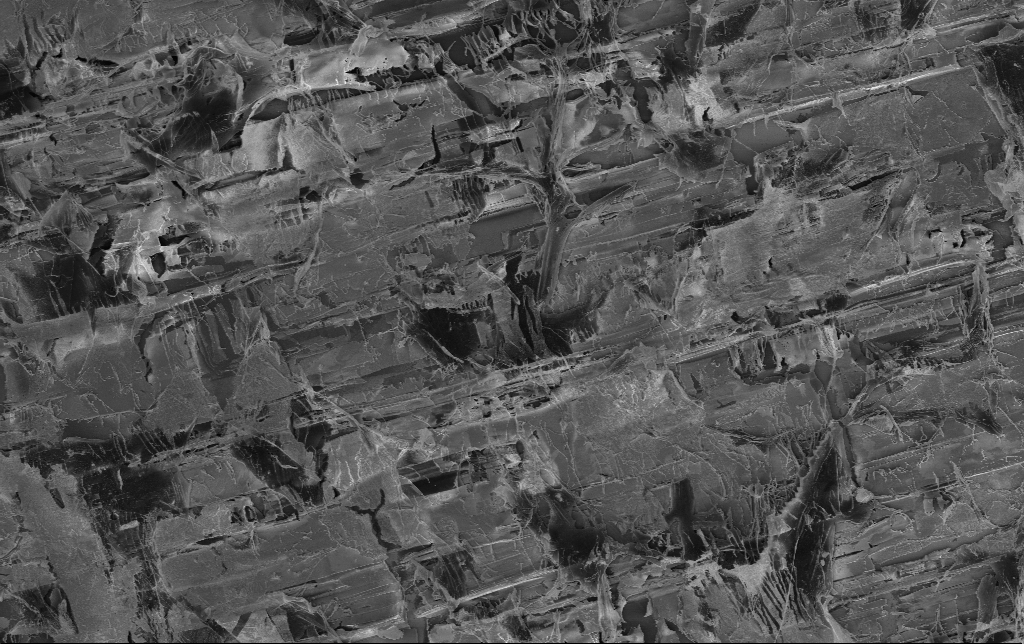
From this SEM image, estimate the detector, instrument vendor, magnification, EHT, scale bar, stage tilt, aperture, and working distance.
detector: InLens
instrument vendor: Zeiss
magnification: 2.49 K X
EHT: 1.5 kV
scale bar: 10000 nm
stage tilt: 0°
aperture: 30 µm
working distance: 3.4 mm